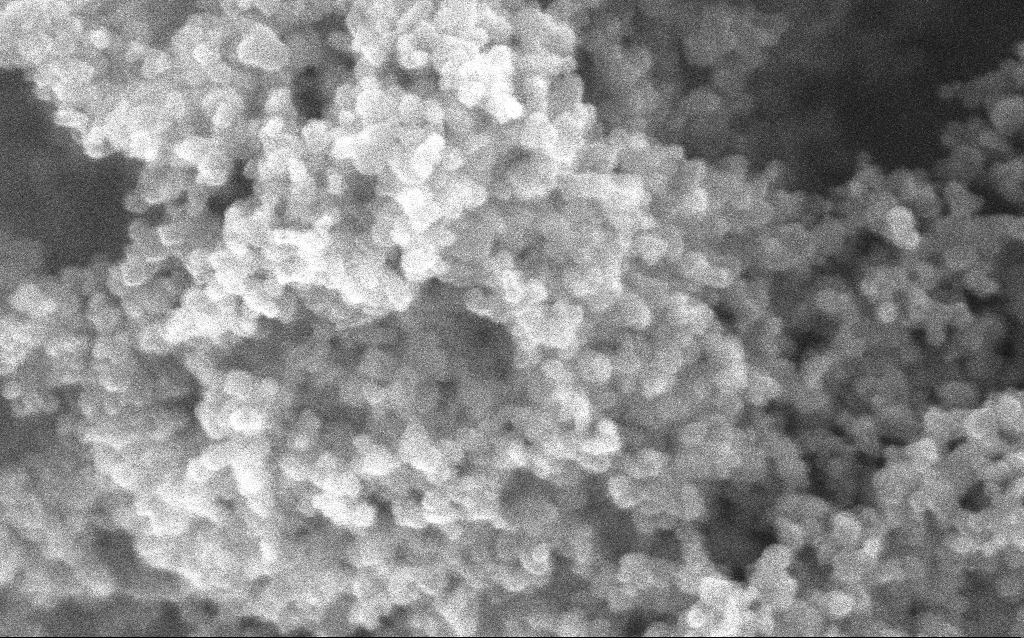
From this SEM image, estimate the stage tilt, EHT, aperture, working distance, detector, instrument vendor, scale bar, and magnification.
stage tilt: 0°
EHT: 10 kV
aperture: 30 µm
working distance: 2.4 mm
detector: InLens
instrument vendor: Zeiss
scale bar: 100 nm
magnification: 348.1 K X